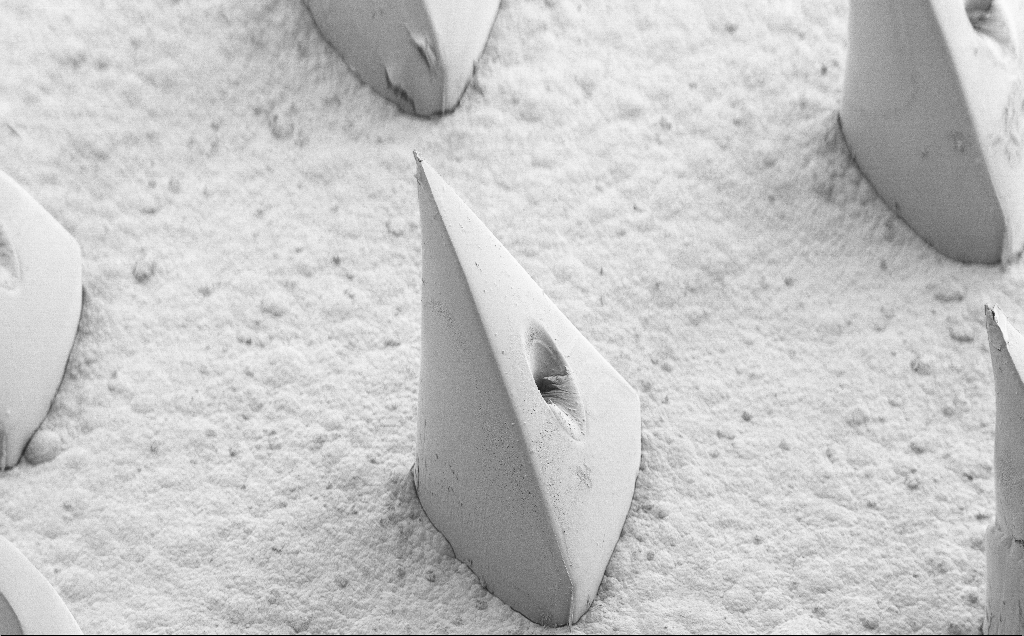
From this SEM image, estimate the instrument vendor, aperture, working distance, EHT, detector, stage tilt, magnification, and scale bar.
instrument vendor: Zeiss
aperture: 30 µm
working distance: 8 mm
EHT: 5 kV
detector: SE2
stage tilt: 40°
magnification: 0.147 K X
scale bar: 100000 nm